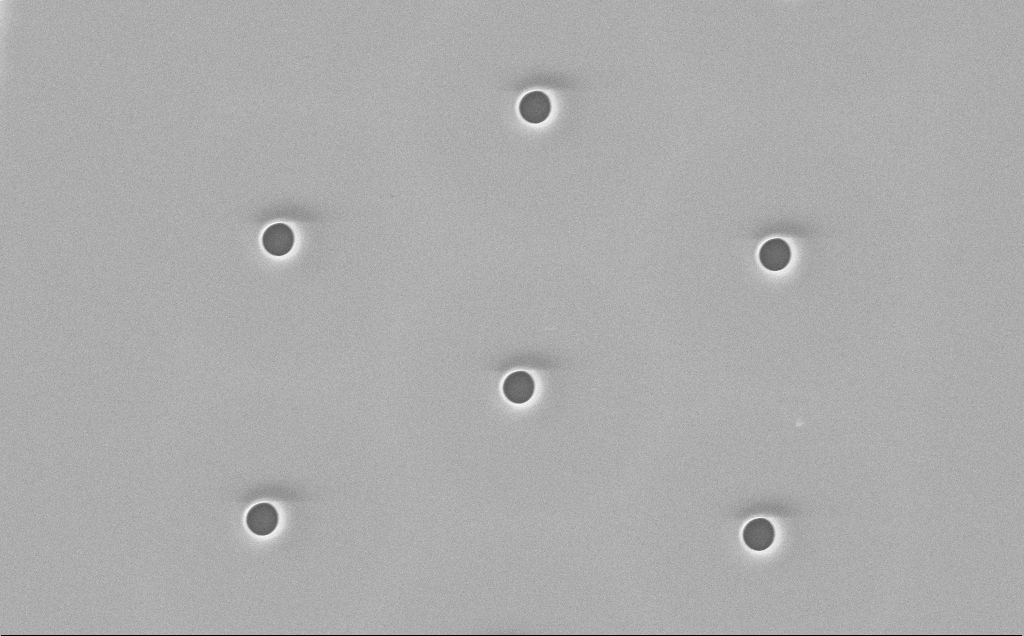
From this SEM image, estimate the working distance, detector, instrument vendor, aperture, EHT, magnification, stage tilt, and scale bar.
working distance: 16 mm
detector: InLens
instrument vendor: Zeiss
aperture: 30 µm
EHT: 10 kV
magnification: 5.13 K X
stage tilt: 0°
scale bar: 10000 nm